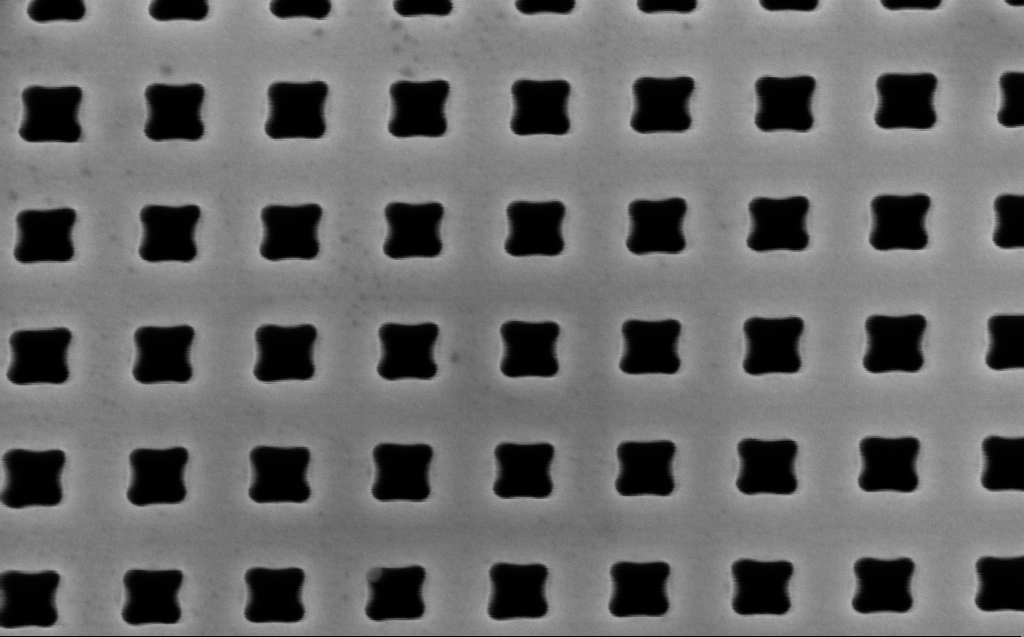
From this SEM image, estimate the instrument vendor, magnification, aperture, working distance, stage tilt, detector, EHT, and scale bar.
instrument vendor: Zeiss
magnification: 90 K X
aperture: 30 µm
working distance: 6 mm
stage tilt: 0°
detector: InLens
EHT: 3 kV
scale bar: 200 nm